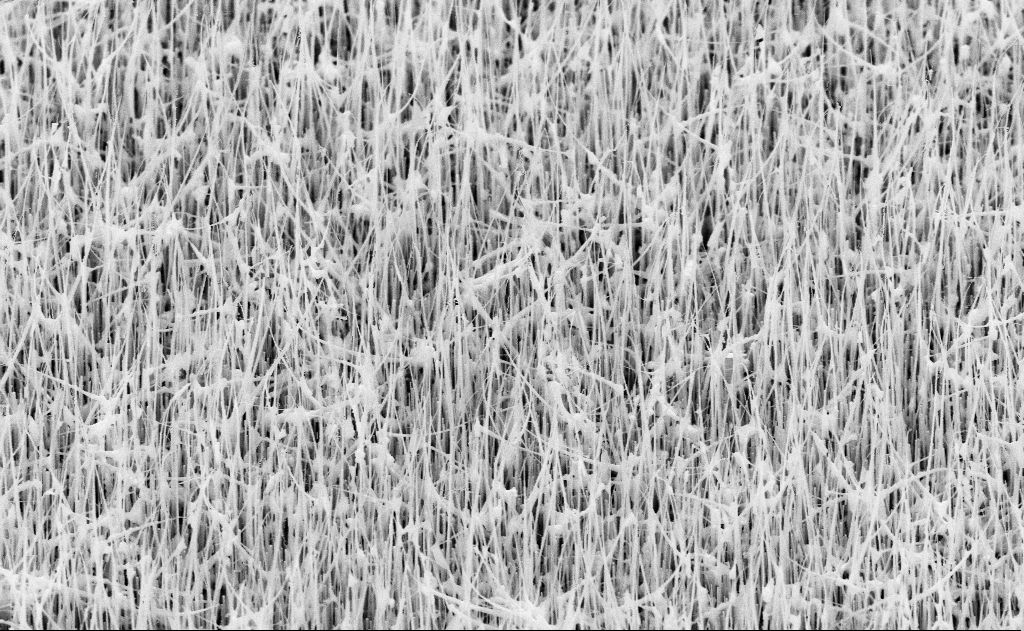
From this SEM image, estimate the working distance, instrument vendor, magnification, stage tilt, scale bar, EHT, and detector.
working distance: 14 mm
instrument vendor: Zeiss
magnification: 20 K X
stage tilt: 45°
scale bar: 2000 nm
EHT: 10 kV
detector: SE2